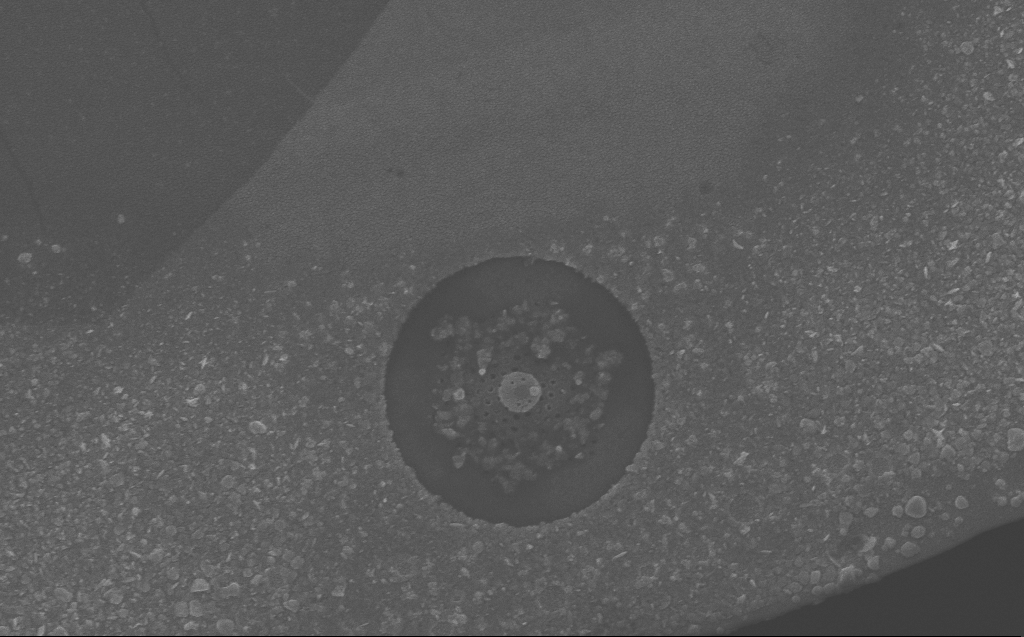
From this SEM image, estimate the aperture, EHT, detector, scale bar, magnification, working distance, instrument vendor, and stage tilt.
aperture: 30 µm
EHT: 10 kV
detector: InLens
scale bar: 2000 nm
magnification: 16.74 K X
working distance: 6 mm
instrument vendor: Zeiss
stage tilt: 0°